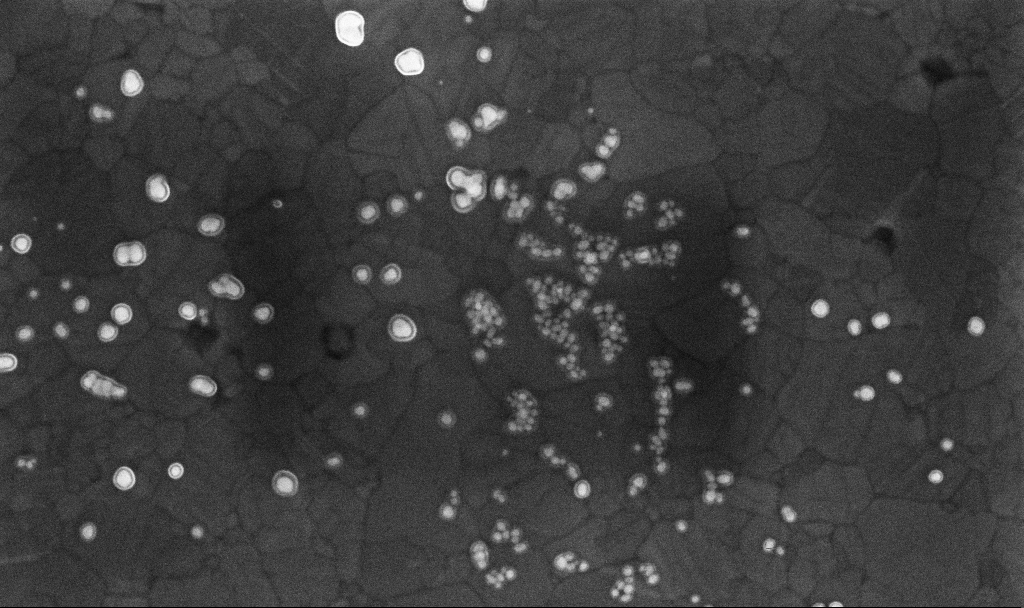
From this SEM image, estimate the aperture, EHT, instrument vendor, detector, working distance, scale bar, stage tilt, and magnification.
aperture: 30 µm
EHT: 10 kV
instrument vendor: Zeiss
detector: InLens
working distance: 3.4 mm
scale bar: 200 nm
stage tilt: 0°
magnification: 128.55 K X